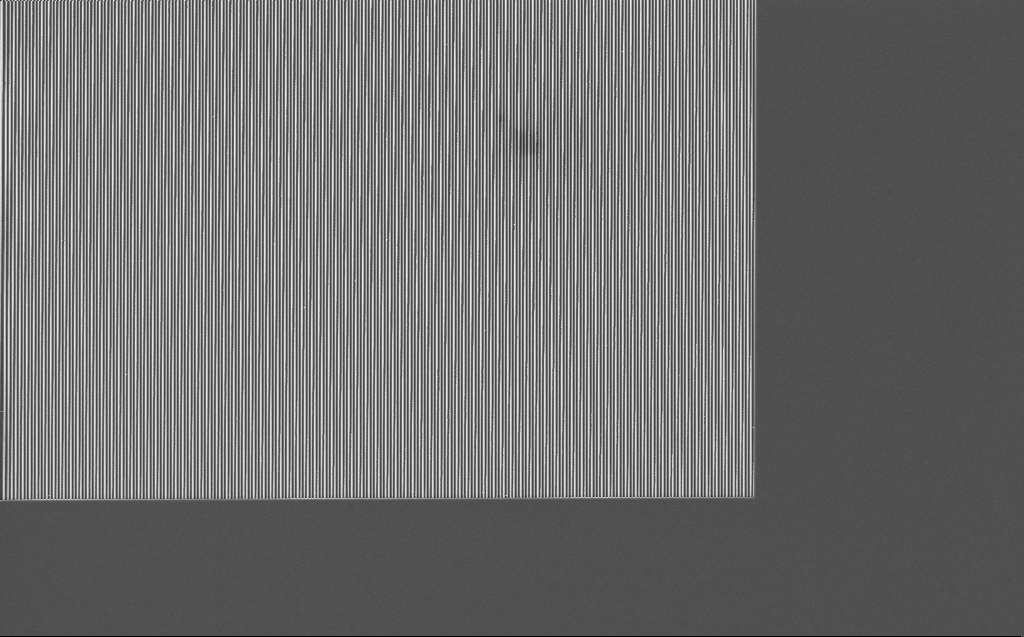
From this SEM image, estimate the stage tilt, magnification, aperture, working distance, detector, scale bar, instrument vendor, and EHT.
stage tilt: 0°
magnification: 3.11 K X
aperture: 30 µm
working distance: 7 mm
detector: InLens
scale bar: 10000 nm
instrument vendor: Zeiss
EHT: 5 kV